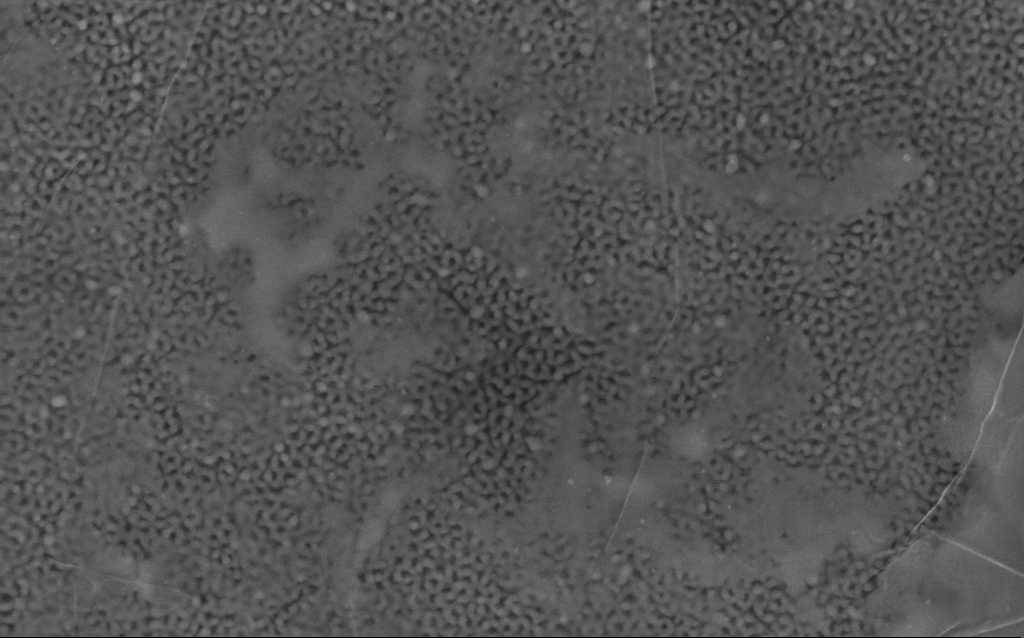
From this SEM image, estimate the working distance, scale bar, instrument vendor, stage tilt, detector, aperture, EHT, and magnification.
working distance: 3.7 mm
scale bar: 100 nm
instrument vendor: Zeiss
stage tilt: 0.1°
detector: InLens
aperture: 30 µm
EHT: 5 kV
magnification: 140.17 K X